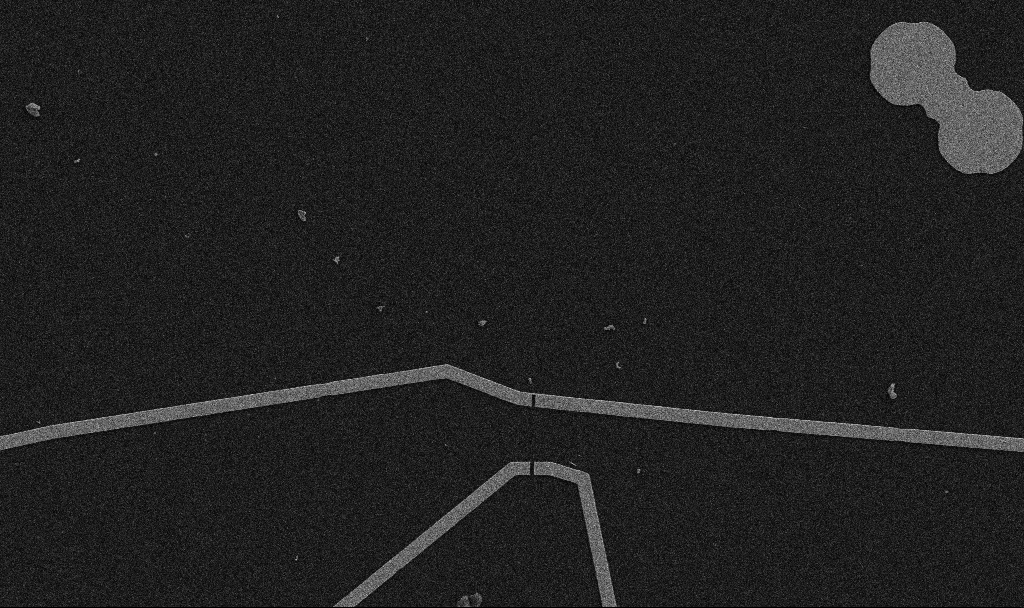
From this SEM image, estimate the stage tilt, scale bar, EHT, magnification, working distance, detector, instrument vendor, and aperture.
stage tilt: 0°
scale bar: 10000 nm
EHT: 5 kV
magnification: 5 K X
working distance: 10.7 mm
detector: SE2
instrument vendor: Zeiss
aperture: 30 µm